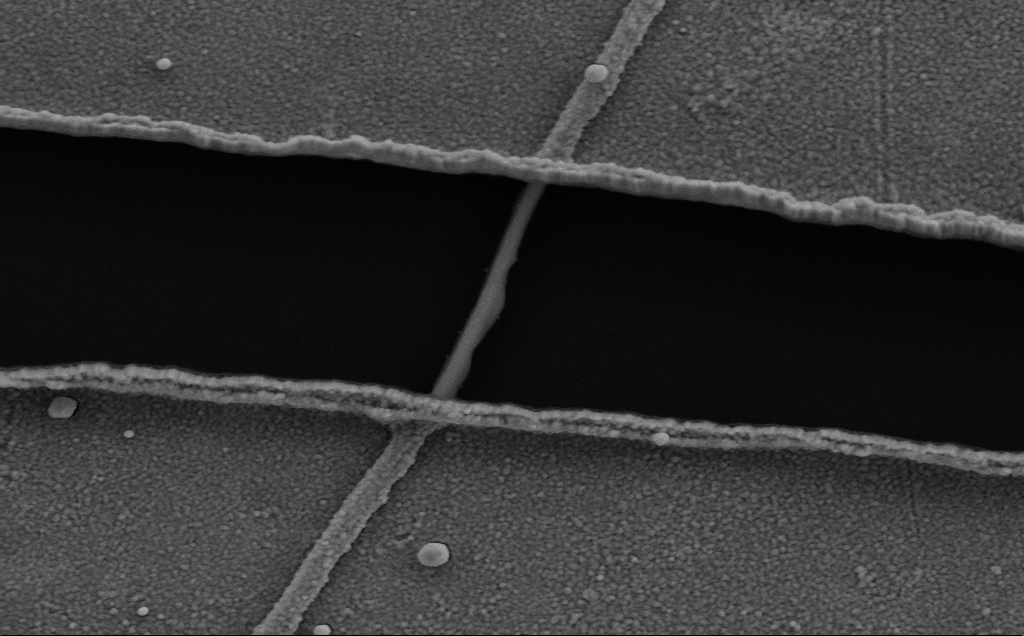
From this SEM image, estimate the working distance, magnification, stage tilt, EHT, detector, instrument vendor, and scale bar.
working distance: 6 mm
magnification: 63.97 K X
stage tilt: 0°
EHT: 5 kV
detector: SE2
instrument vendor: Zeiss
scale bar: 1000 nm